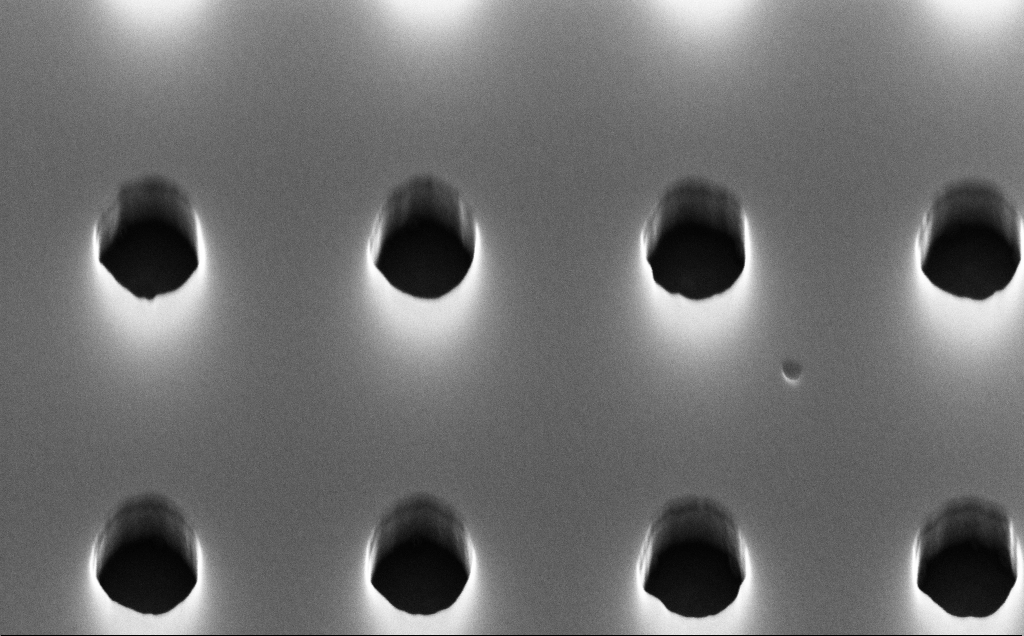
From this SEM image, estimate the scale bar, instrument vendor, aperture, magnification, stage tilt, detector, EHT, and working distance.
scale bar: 200 nm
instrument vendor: Zeiss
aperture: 30 µm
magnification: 95.95 K X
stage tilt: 45°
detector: InLens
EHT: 10 kV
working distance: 3 mm